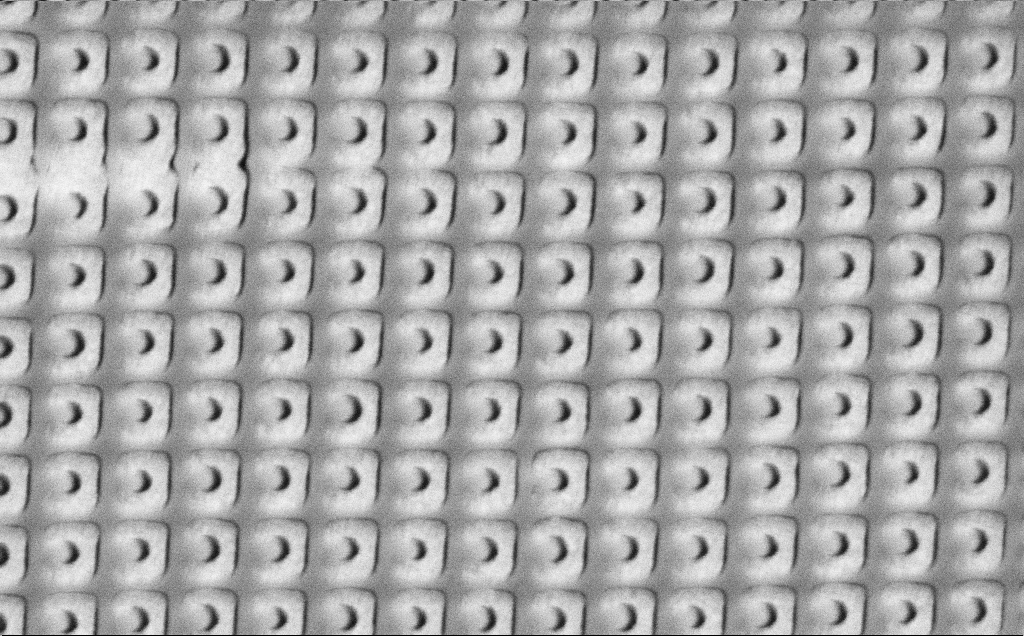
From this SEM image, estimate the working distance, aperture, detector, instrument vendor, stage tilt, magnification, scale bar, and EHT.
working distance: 8.3 mm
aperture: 30 µm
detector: SE2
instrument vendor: Zeiss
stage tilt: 0°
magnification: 54.96 K X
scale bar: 1000 nm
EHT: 3 kV